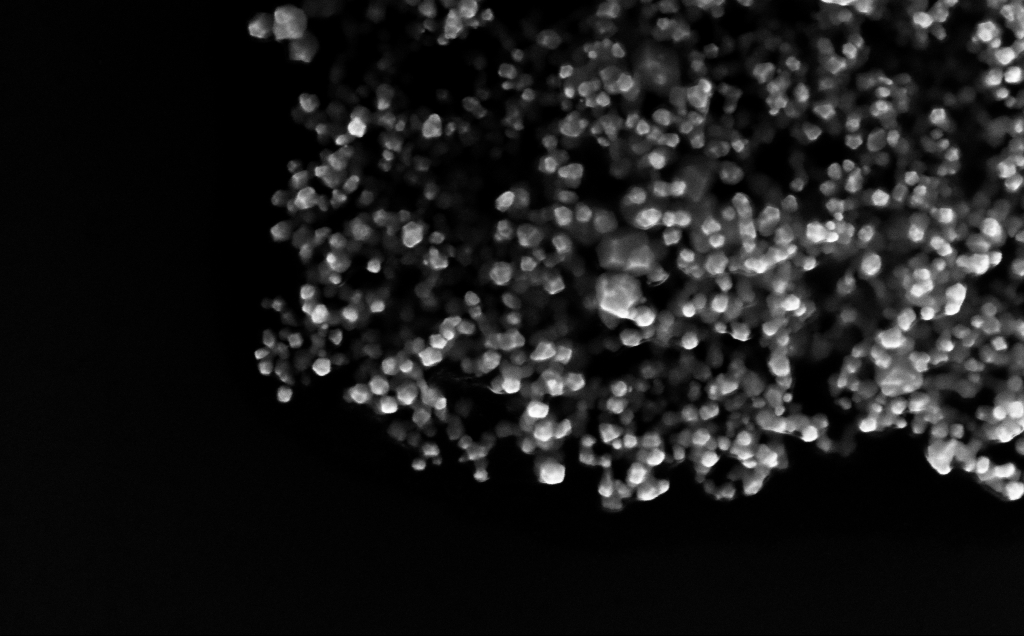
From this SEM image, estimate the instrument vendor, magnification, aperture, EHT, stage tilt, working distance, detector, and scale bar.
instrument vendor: Zeiss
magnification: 165.95 K X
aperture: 30 µm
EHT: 10 kV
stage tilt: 0°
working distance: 4 mm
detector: InLens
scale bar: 100 nm